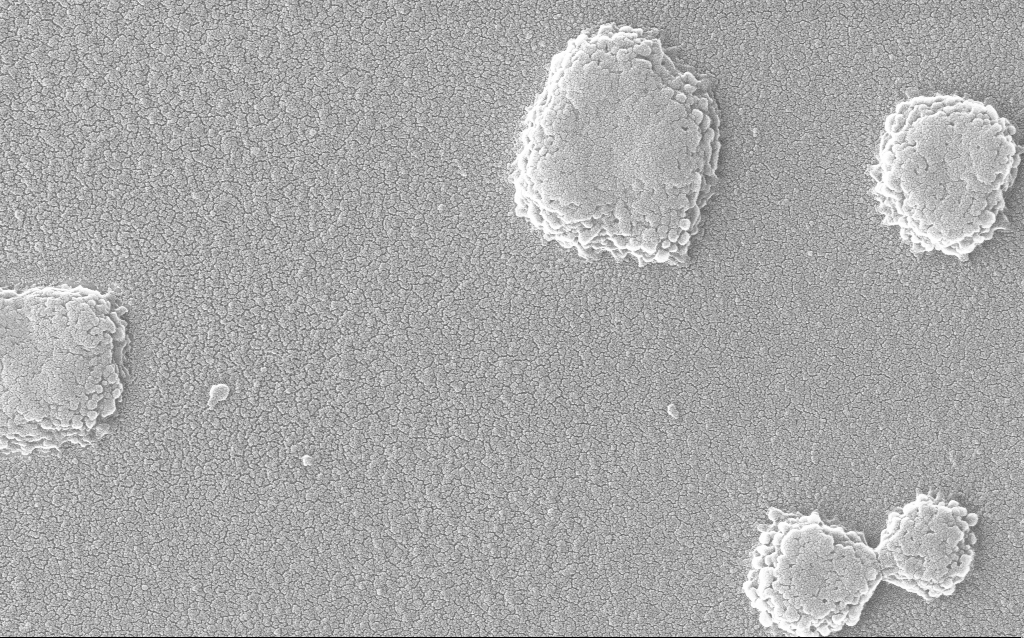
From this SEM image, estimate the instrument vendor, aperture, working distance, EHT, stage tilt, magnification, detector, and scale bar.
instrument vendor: Zeiss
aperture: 30 µm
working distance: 1.8 mm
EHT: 20 kV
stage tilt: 0°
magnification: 100 K X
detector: InLens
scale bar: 200 nm